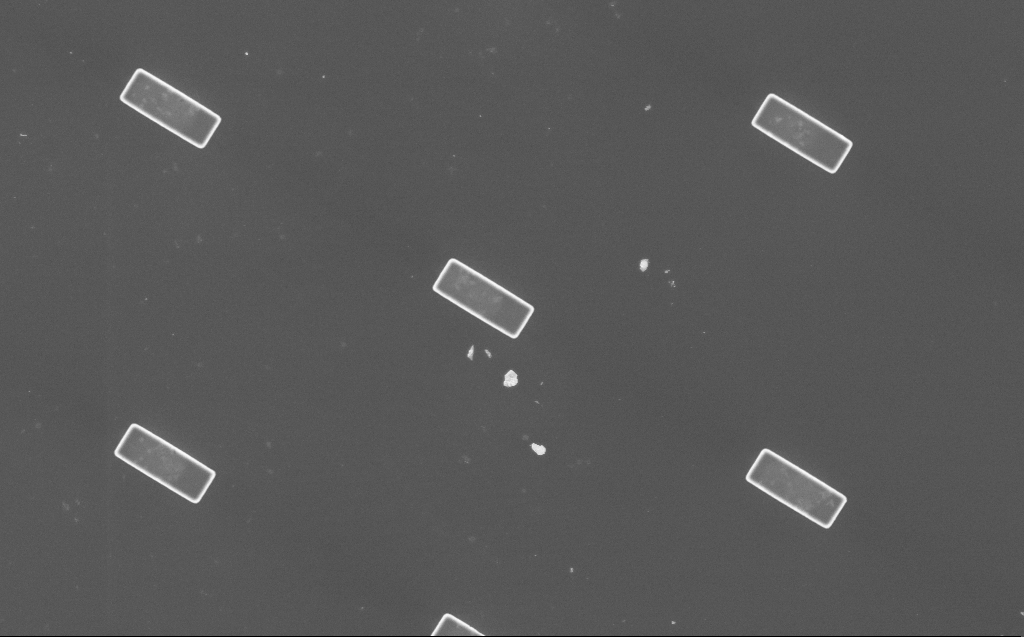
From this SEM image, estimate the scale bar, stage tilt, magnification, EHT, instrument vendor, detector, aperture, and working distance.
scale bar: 10000 nm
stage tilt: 0°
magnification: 4.73 K X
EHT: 5 kV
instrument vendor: Zeiss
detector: InLens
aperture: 30 µm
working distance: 8 mm